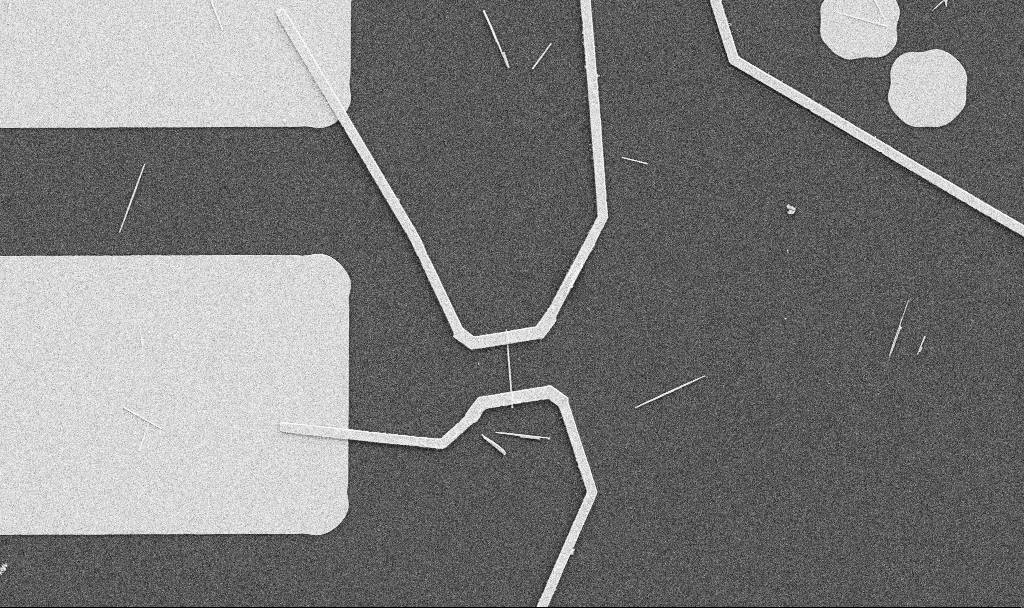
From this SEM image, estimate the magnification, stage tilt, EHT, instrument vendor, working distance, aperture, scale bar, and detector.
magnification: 5 K X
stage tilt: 0°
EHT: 5 kV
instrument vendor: Zeiss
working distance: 10.7 mm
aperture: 30 µm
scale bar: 10000 nm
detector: SE2